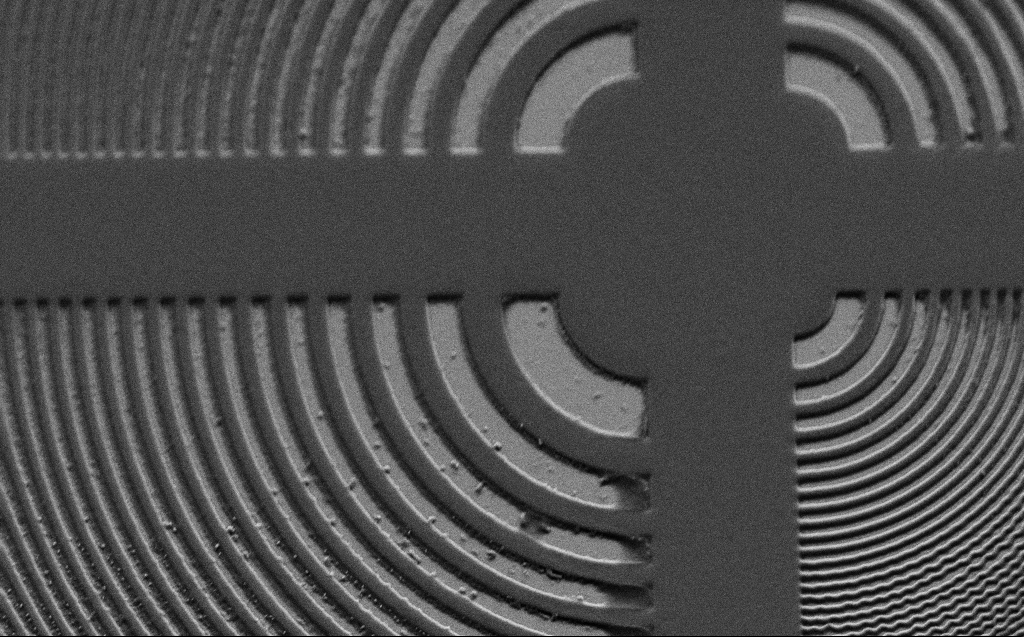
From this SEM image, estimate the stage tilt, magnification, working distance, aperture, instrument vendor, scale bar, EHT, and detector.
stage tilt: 45°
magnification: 5.54 K X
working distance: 6 mm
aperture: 30 µm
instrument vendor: Zeiss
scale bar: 10000 nm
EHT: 3 kV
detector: SE2